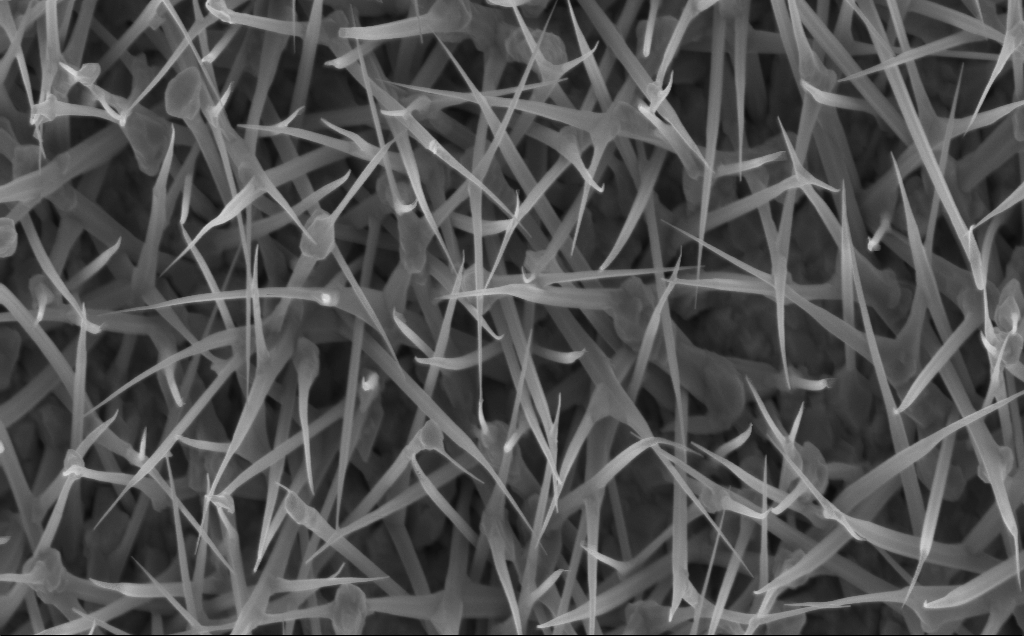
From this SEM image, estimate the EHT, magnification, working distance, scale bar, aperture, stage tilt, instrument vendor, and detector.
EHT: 5 kV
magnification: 40 K X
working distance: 5 mm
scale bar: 1000 nm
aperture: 30 µm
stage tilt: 0°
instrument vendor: Zeiss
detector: InLens